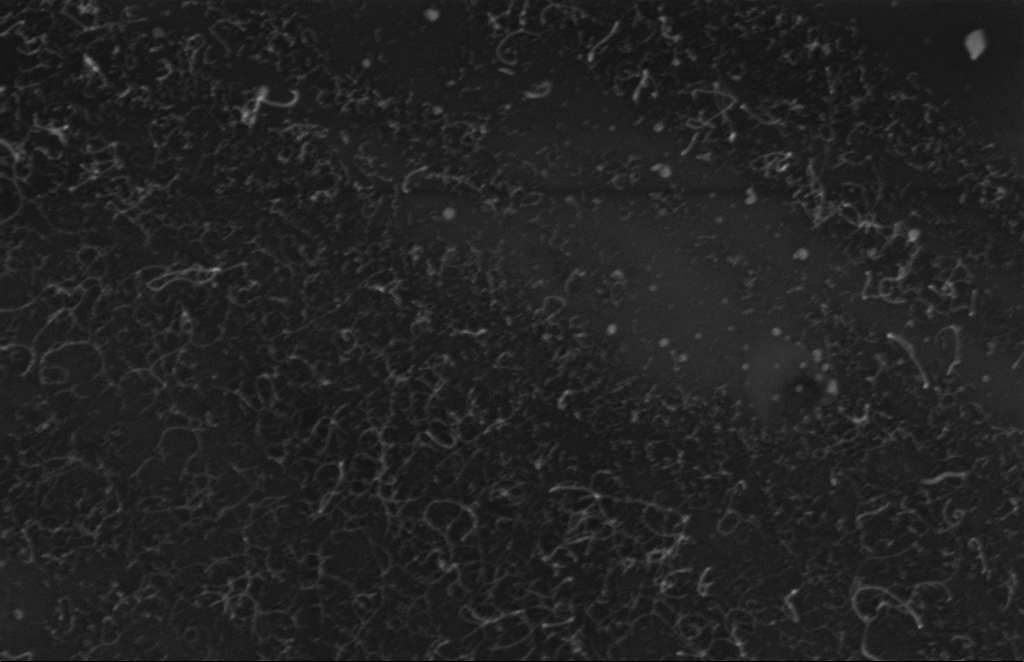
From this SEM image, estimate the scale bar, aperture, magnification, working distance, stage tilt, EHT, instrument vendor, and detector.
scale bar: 200 nm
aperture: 20 µm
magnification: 114.1 K X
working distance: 5 mm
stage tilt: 0°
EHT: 5 kV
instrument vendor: Zeiss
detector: InLens